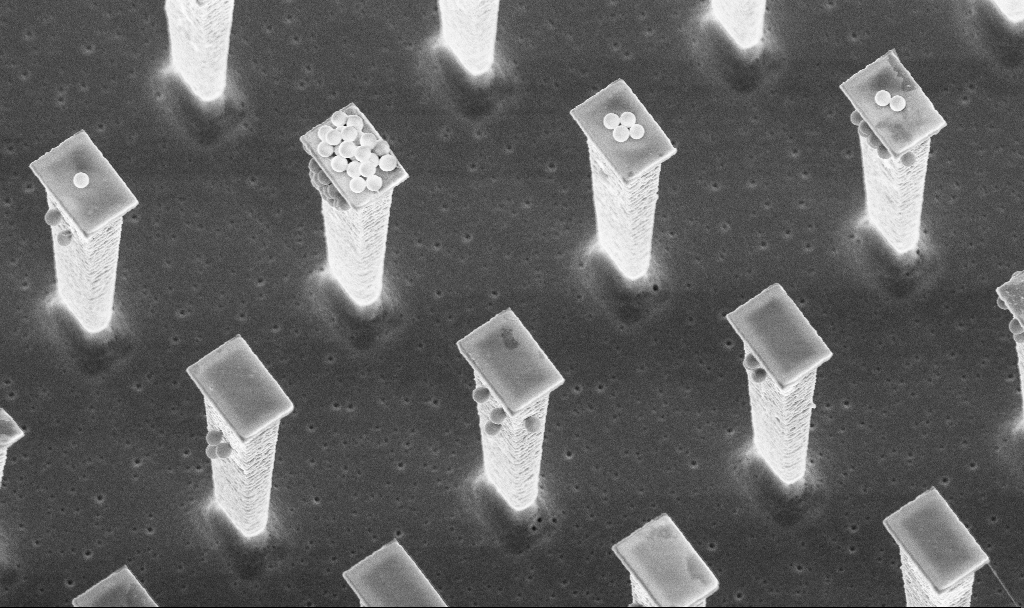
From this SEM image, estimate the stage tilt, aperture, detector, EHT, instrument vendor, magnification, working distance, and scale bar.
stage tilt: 20°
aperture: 30 µm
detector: InLens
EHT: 5 kV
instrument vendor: Zeiss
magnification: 8.24 K X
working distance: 4.8 mm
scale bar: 2000 nm